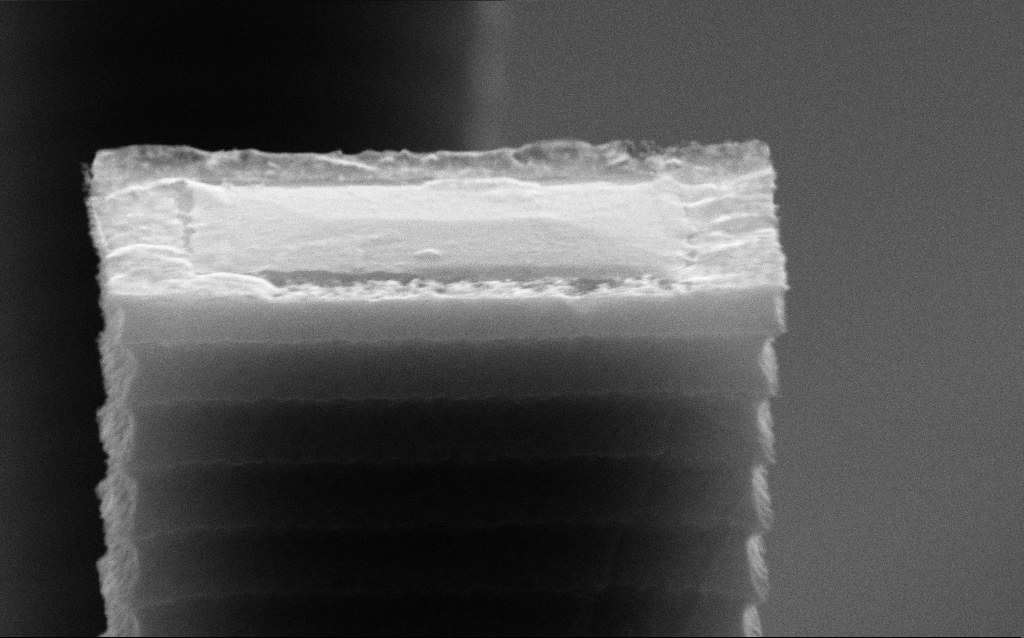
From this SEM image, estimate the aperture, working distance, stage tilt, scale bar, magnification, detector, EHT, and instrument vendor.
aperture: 30 µm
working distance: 7.4 mm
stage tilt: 70°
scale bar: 200 nm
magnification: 85.46 K X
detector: SE2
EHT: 10 kV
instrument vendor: Zeiss